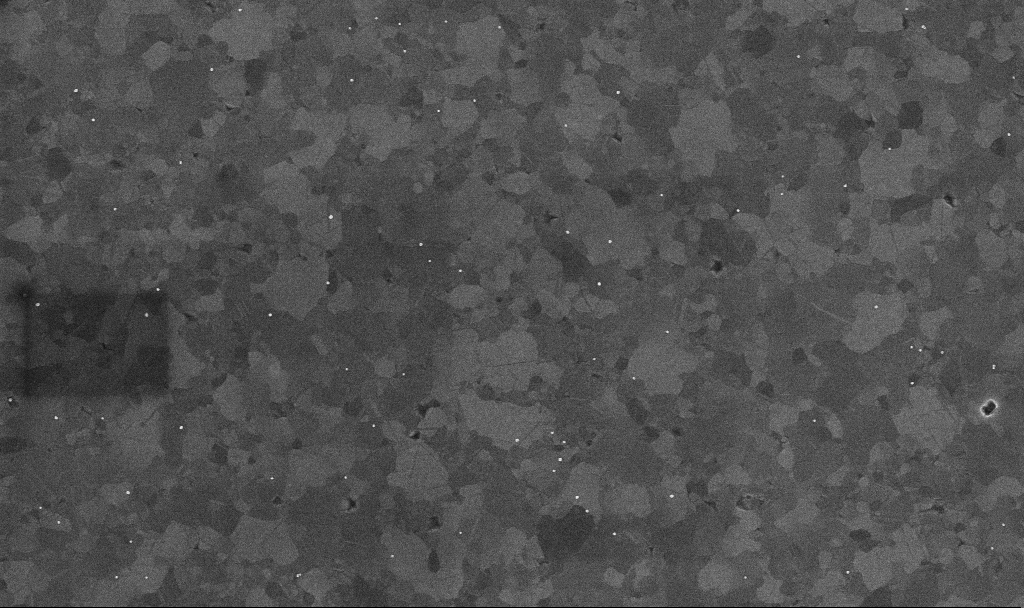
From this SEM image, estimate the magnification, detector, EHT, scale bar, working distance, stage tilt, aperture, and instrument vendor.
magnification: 50 K X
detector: InLens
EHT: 10 kV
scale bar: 1000 nm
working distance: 3.3 mm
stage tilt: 0°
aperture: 30 µm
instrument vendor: Zeiss